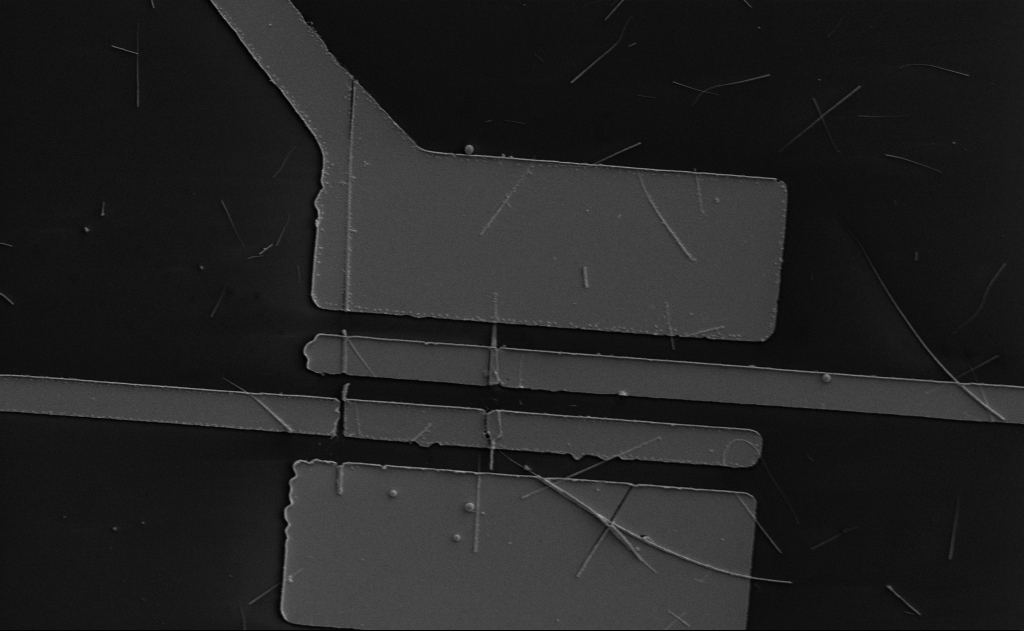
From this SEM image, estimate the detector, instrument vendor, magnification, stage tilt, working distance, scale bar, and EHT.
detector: SE2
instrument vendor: Zeiss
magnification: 5.68 K X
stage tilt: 0°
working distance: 15 mm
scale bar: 10000 nm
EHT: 5 kV